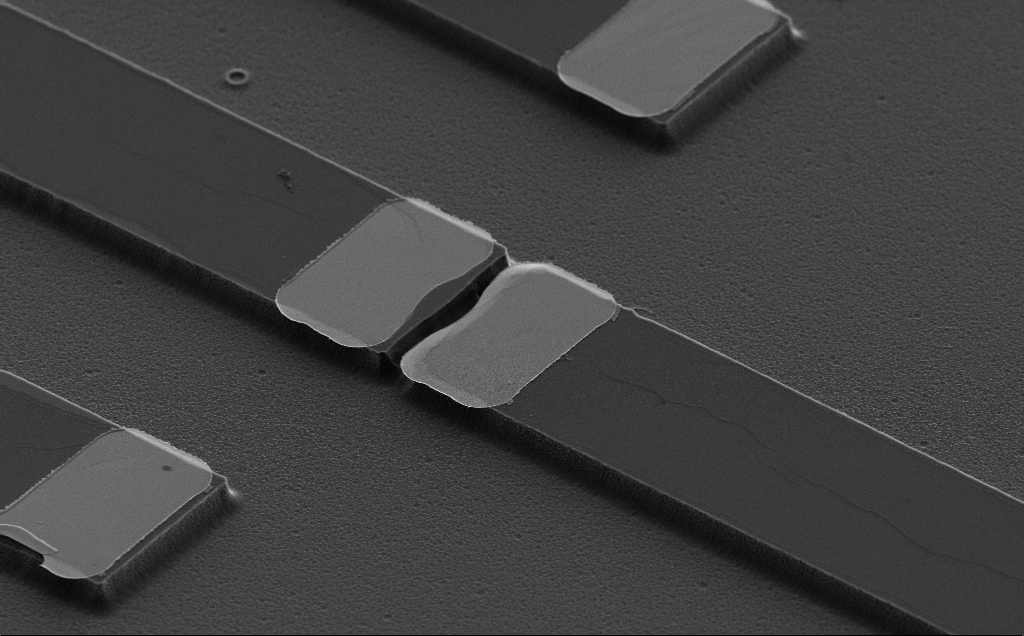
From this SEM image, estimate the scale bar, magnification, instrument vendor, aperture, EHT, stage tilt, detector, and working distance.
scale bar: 10000 nm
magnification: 4.23 K X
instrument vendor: Zeiss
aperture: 30 µm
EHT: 5 kV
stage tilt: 50°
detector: SE2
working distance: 10 mm